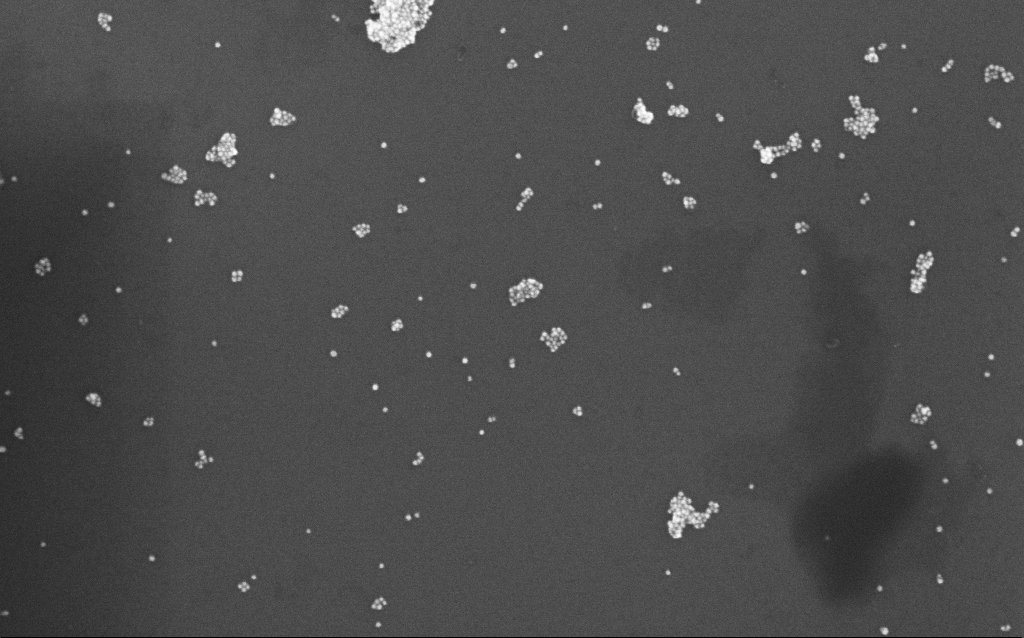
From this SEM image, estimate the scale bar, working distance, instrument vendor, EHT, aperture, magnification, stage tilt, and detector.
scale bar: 200 nm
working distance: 7 mm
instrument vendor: Zeiss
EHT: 10 kV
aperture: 30 µm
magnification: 100 K X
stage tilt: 0°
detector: InLens